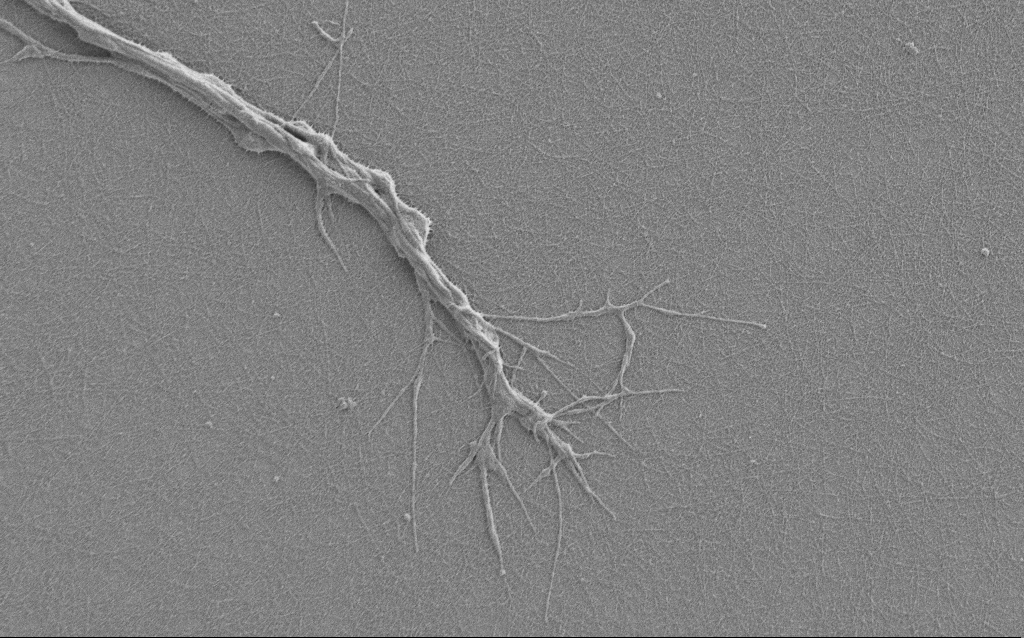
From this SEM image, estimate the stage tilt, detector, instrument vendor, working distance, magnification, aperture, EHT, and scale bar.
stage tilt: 0°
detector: SE2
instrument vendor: Zeiss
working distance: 6 mm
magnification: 7.5 K X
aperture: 30 µm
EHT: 1 kV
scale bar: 2000 nm